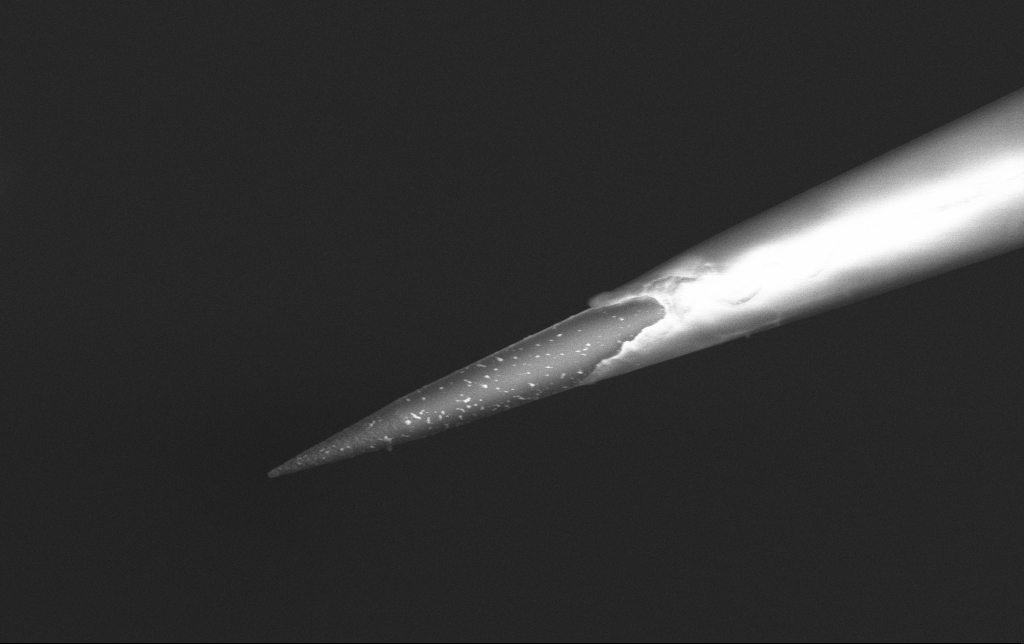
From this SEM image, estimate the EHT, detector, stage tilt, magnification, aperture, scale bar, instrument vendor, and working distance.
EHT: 3 kV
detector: InLens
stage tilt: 0°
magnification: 10 K X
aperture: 30 µm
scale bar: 2000 nm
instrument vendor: Zeiss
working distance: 6.6 mm